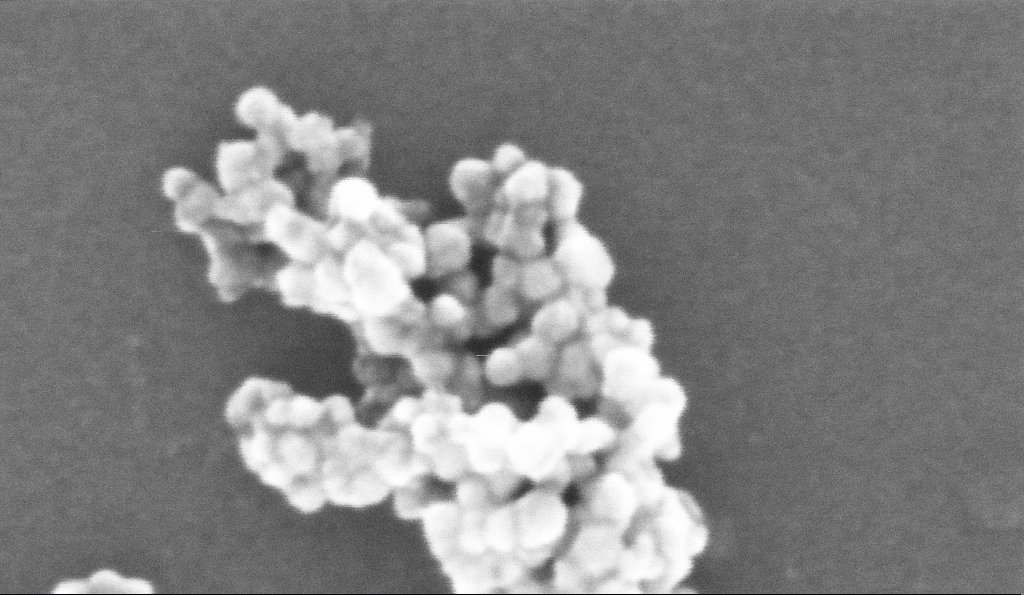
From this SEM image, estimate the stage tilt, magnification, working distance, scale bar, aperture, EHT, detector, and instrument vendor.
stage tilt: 0°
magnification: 787.26 K X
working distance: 5.2 mm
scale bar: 20 nm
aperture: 30 µm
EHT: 10 kV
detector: InLens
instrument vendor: Zeiss